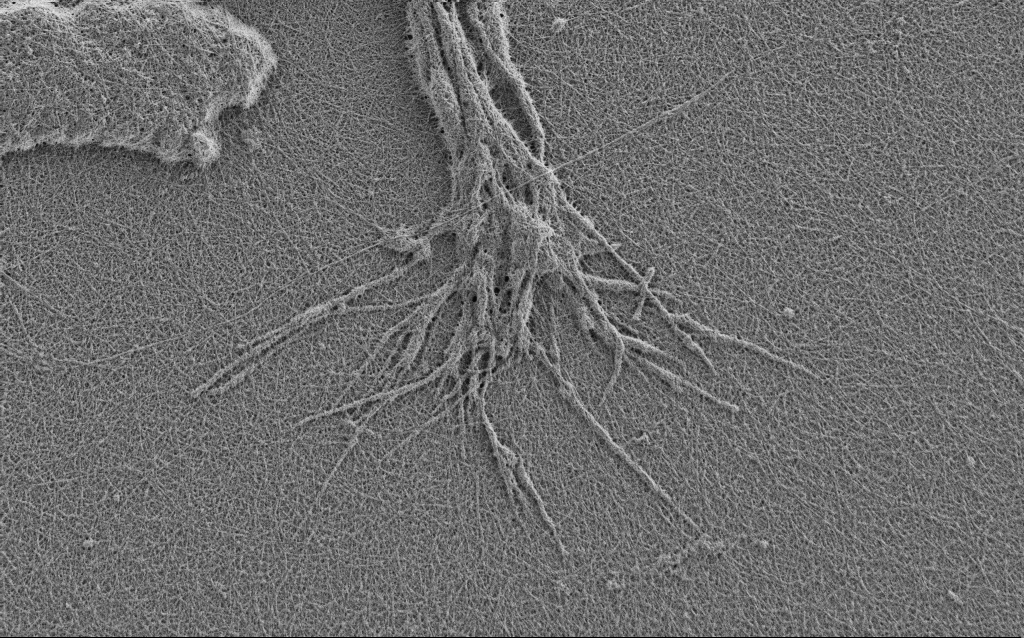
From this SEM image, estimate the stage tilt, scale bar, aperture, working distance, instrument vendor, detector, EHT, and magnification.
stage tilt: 0°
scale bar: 2000 nm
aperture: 30 µm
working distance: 4 mm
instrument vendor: Zeiss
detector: SE2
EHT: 0.9 kV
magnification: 10 K X